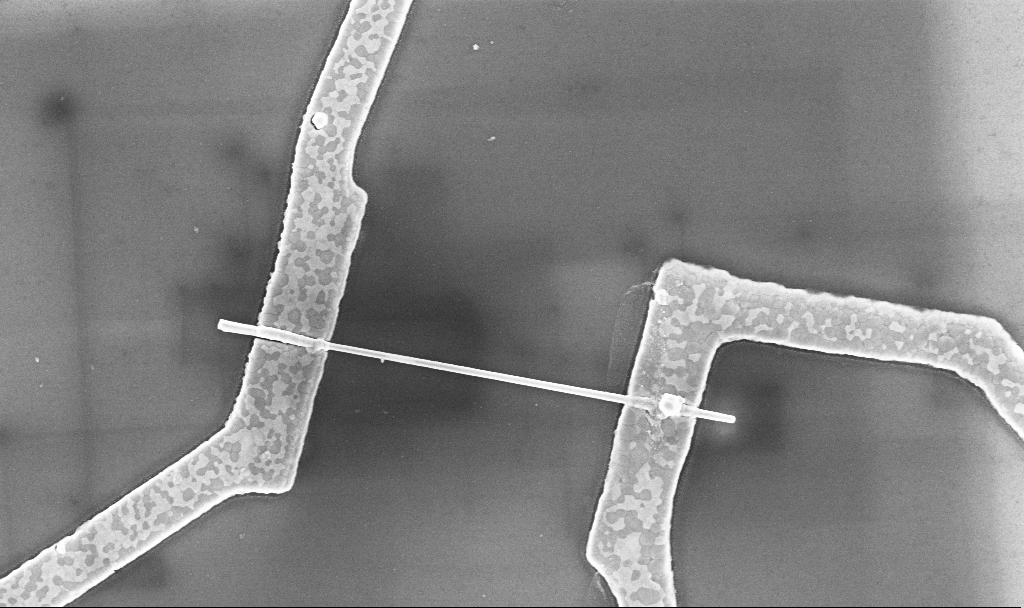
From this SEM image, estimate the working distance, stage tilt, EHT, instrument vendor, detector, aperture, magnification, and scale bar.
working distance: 6.7 mm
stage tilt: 0°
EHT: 5 kV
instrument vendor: Zeiss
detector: InLens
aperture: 30 µm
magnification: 30 K X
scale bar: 2000 nm